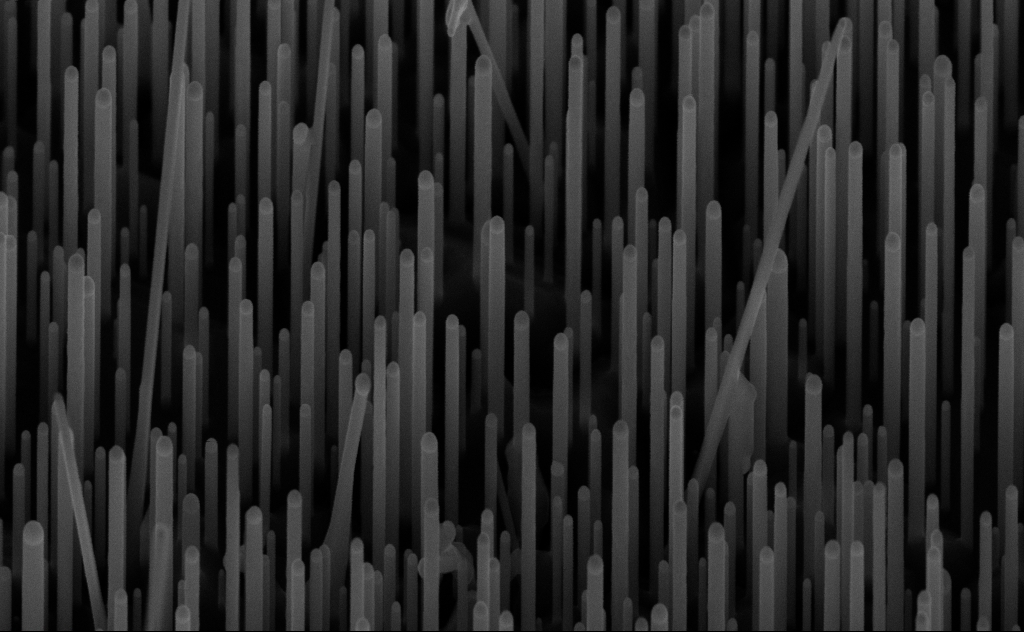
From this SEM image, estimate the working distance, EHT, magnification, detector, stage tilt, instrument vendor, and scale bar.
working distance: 7 mm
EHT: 10 kV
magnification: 92.01 K X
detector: InLens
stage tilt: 45°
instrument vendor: Zeiss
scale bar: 200 nm